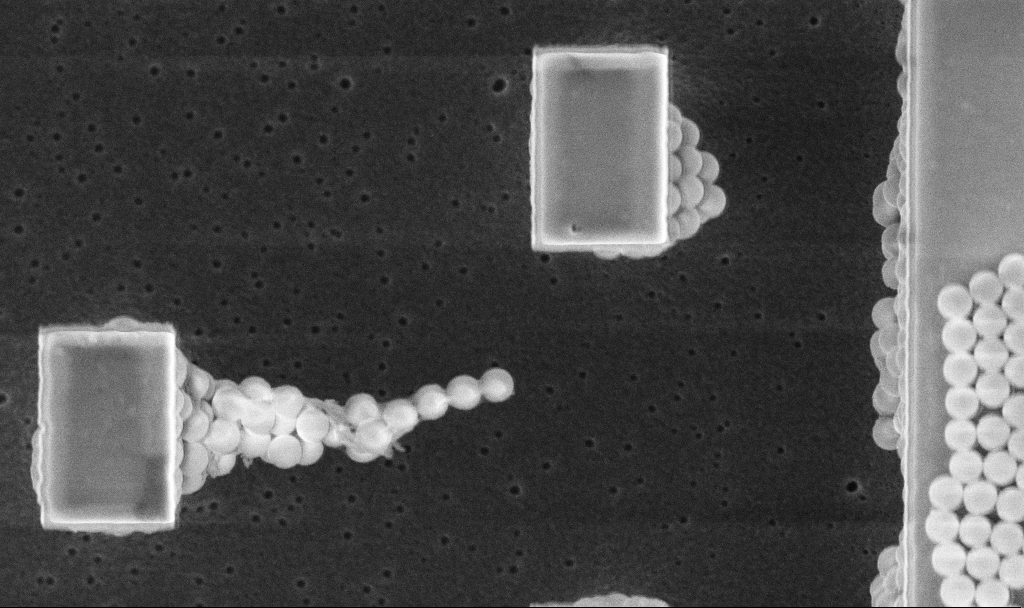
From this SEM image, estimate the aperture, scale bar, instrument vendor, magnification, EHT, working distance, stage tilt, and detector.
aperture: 30 µm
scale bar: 1000 nm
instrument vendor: Zeiss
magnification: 17.18 K X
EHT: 5 kV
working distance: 3.2 mm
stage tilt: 0°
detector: InLens